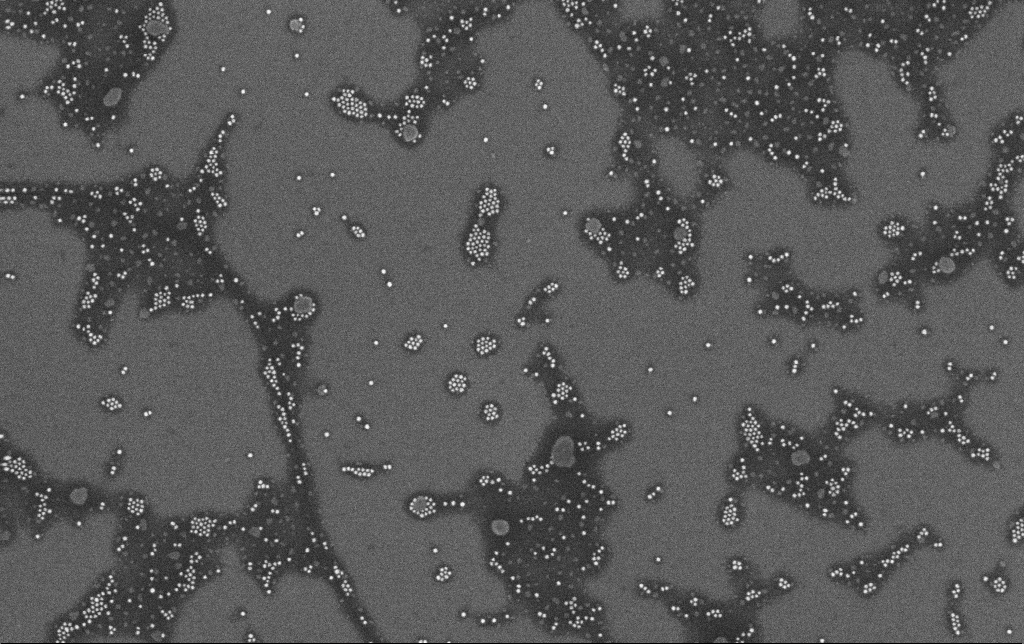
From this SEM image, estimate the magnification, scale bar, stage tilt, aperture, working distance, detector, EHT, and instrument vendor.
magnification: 100 K X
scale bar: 200 nm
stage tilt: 0°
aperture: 30 µm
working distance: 3.4 mm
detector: InLens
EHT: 10 kV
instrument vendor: Zeiss